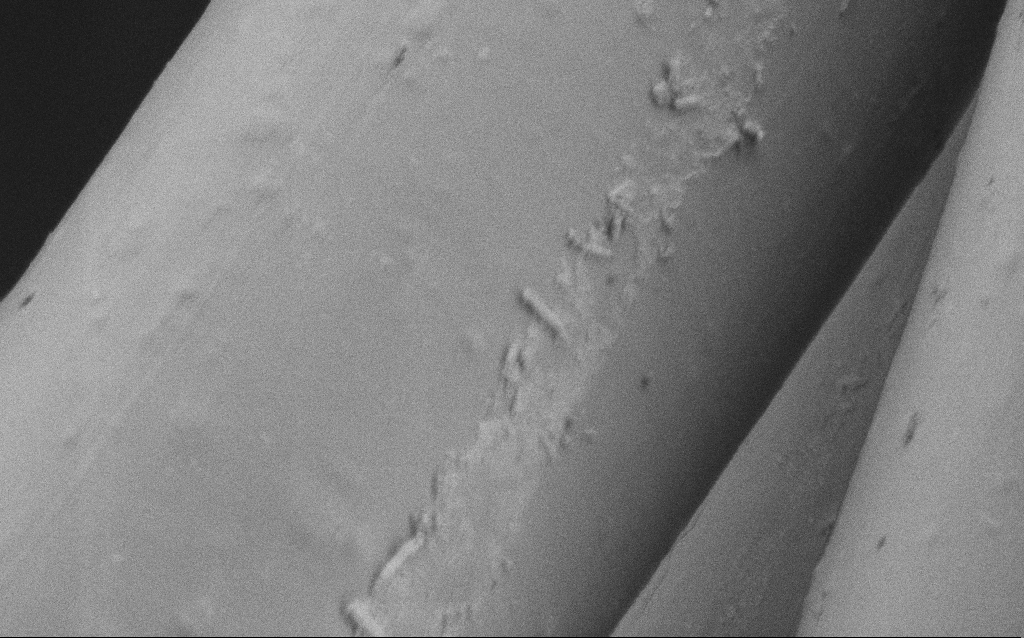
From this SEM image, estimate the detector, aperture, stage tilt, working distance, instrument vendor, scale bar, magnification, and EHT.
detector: SE2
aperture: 30 µm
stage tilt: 0°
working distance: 4 mm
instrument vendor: Zeiss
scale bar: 2000 nm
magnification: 10.67 K X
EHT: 1 kV